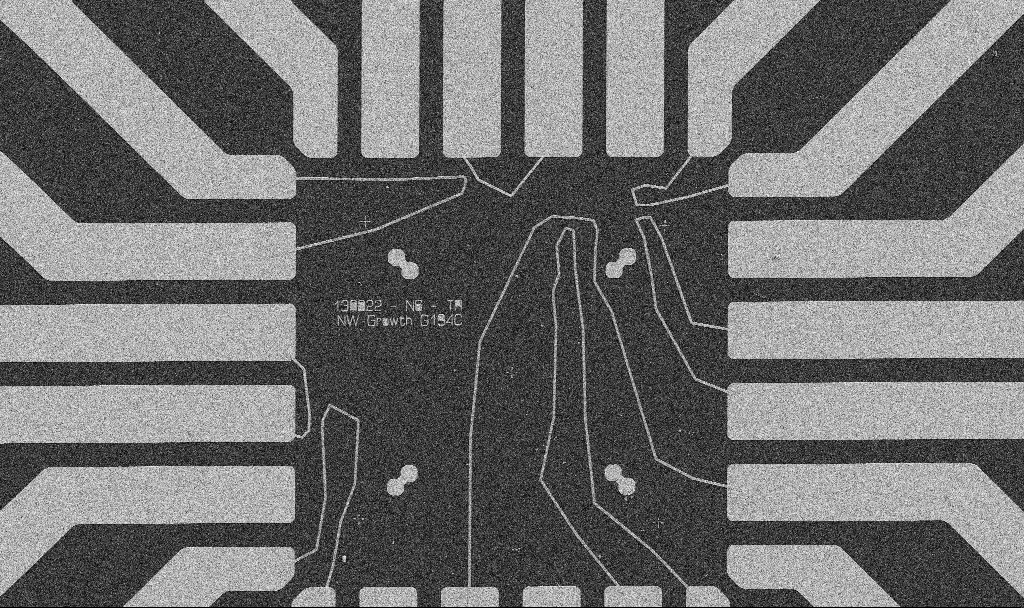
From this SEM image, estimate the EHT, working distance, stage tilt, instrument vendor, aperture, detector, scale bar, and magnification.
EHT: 5 kV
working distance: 8.7 mm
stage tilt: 0°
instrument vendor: Zeiss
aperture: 30 µm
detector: SE2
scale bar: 20000 nm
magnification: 1 K X